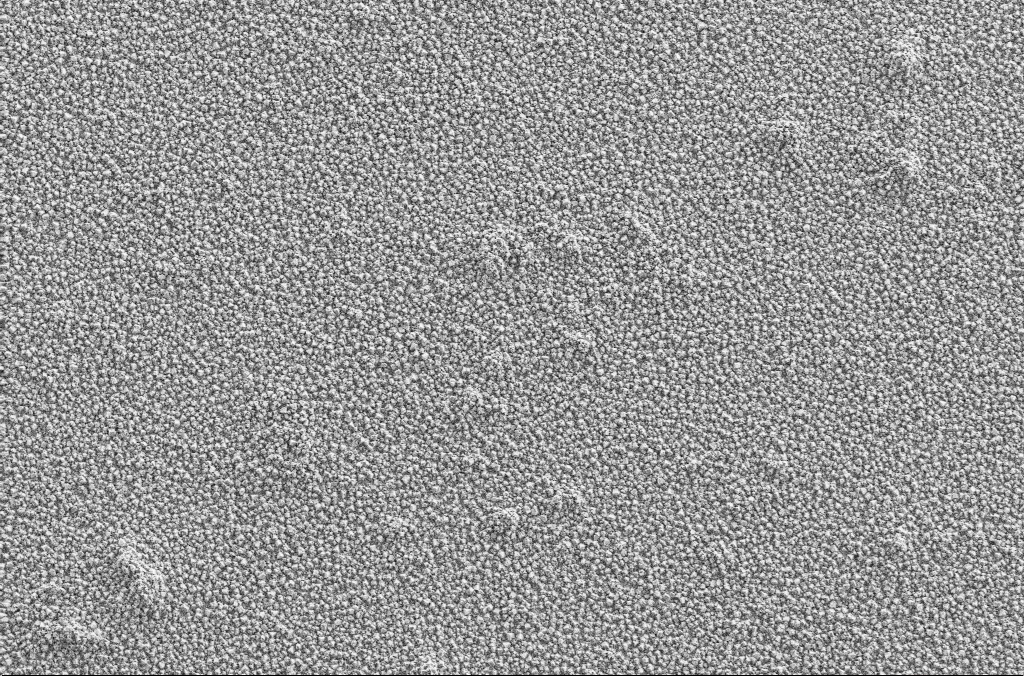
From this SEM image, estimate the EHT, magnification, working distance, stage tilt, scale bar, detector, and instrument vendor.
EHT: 2 kV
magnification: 5 K X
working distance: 1.9 mm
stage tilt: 0°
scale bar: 10000 nm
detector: SE2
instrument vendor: Zeiss